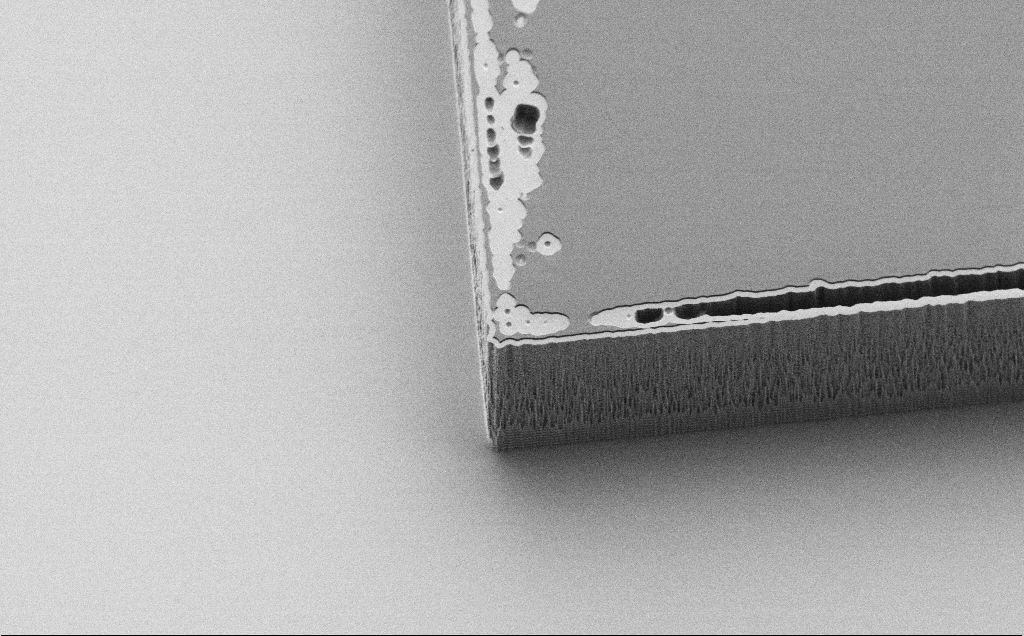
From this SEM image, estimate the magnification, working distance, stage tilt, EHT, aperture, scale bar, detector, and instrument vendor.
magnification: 2.53 K X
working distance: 7 mm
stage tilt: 45°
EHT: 1.2 kV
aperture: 30 µm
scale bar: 20000 nm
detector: SE2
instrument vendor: Zeiss